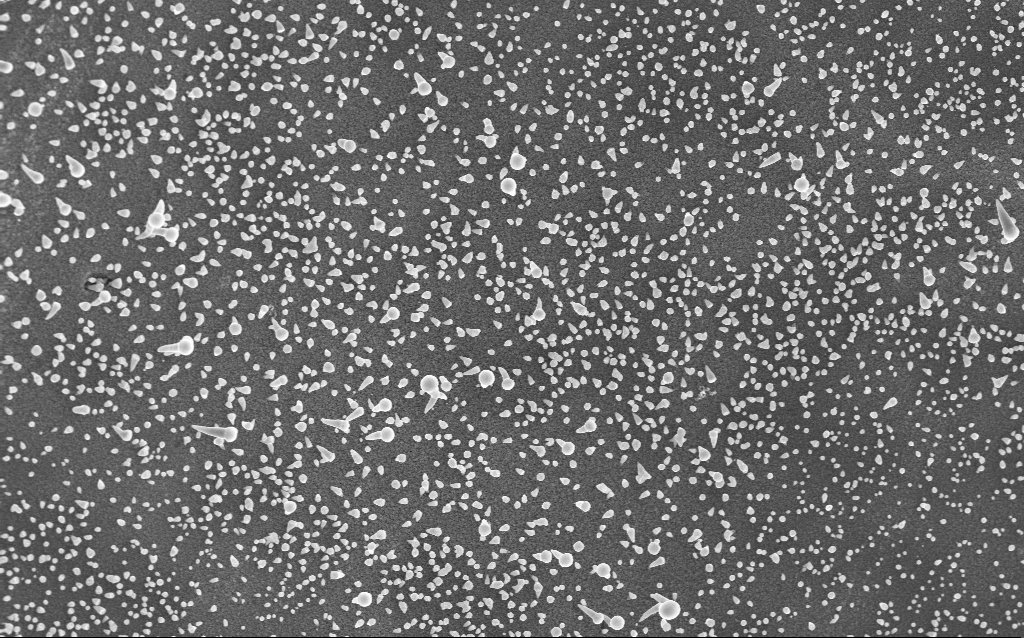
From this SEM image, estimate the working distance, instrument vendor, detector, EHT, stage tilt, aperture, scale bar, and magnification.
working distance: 4 mm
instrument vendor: Zeiss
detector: InLens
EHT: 5 kV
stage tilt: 0°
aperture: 30 µm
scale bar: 1000 nm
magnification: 20 K X